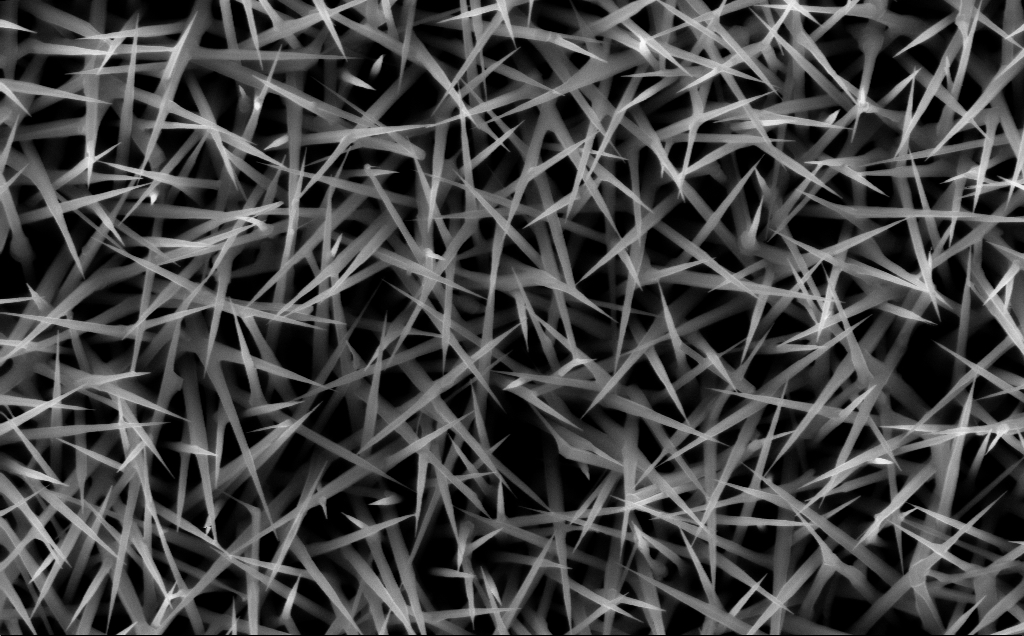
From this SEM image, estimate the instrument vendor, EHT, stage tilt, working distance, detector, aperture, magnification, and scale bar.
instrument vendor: Zeiss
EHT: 10 kV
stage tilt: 0°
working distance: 7 mm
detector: InLens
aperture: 30 µm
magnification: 40 K X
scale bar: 1000 nm